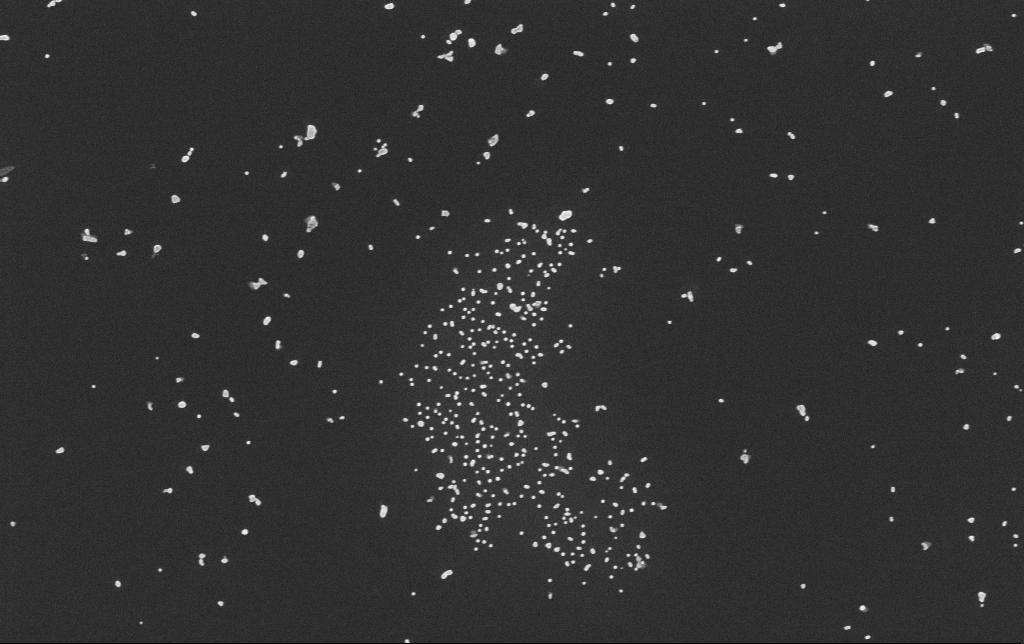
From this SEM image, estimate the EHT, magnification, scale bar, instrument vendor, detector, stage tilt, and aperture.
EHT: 10 kV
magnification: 50 K X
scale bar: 1000 nm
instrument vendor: Zeiss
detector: InLens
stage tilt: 0°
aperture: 30 µm